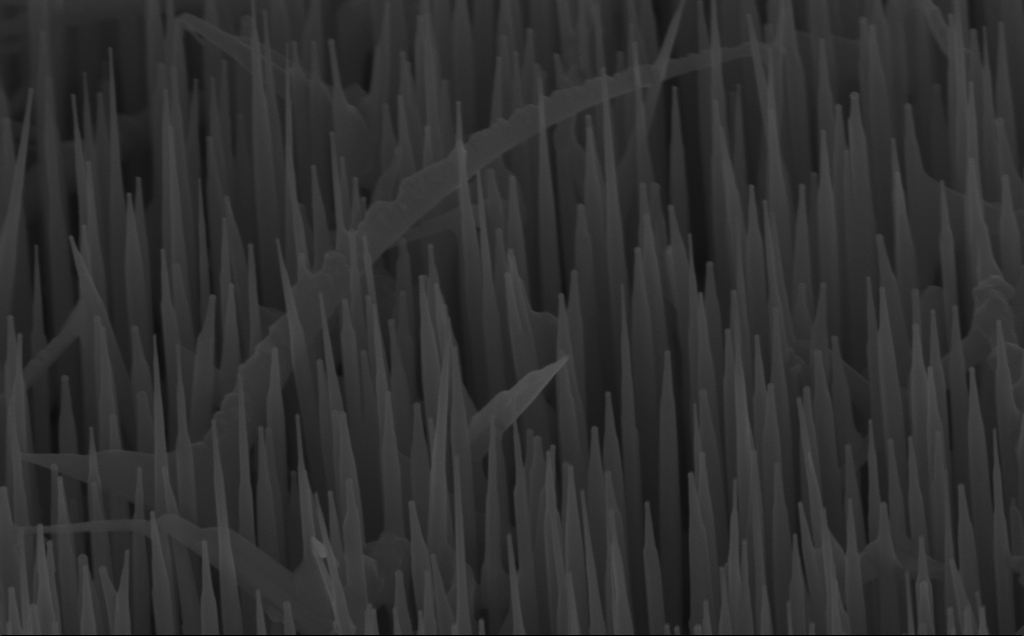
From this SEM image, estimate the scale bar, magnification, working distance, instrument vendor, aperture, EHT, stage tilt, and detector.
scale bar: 200 nm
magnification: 80 K X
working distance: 6 mm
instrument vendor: Zeiss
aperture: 30 µm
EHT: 10 kV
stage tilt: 45°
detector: InLens